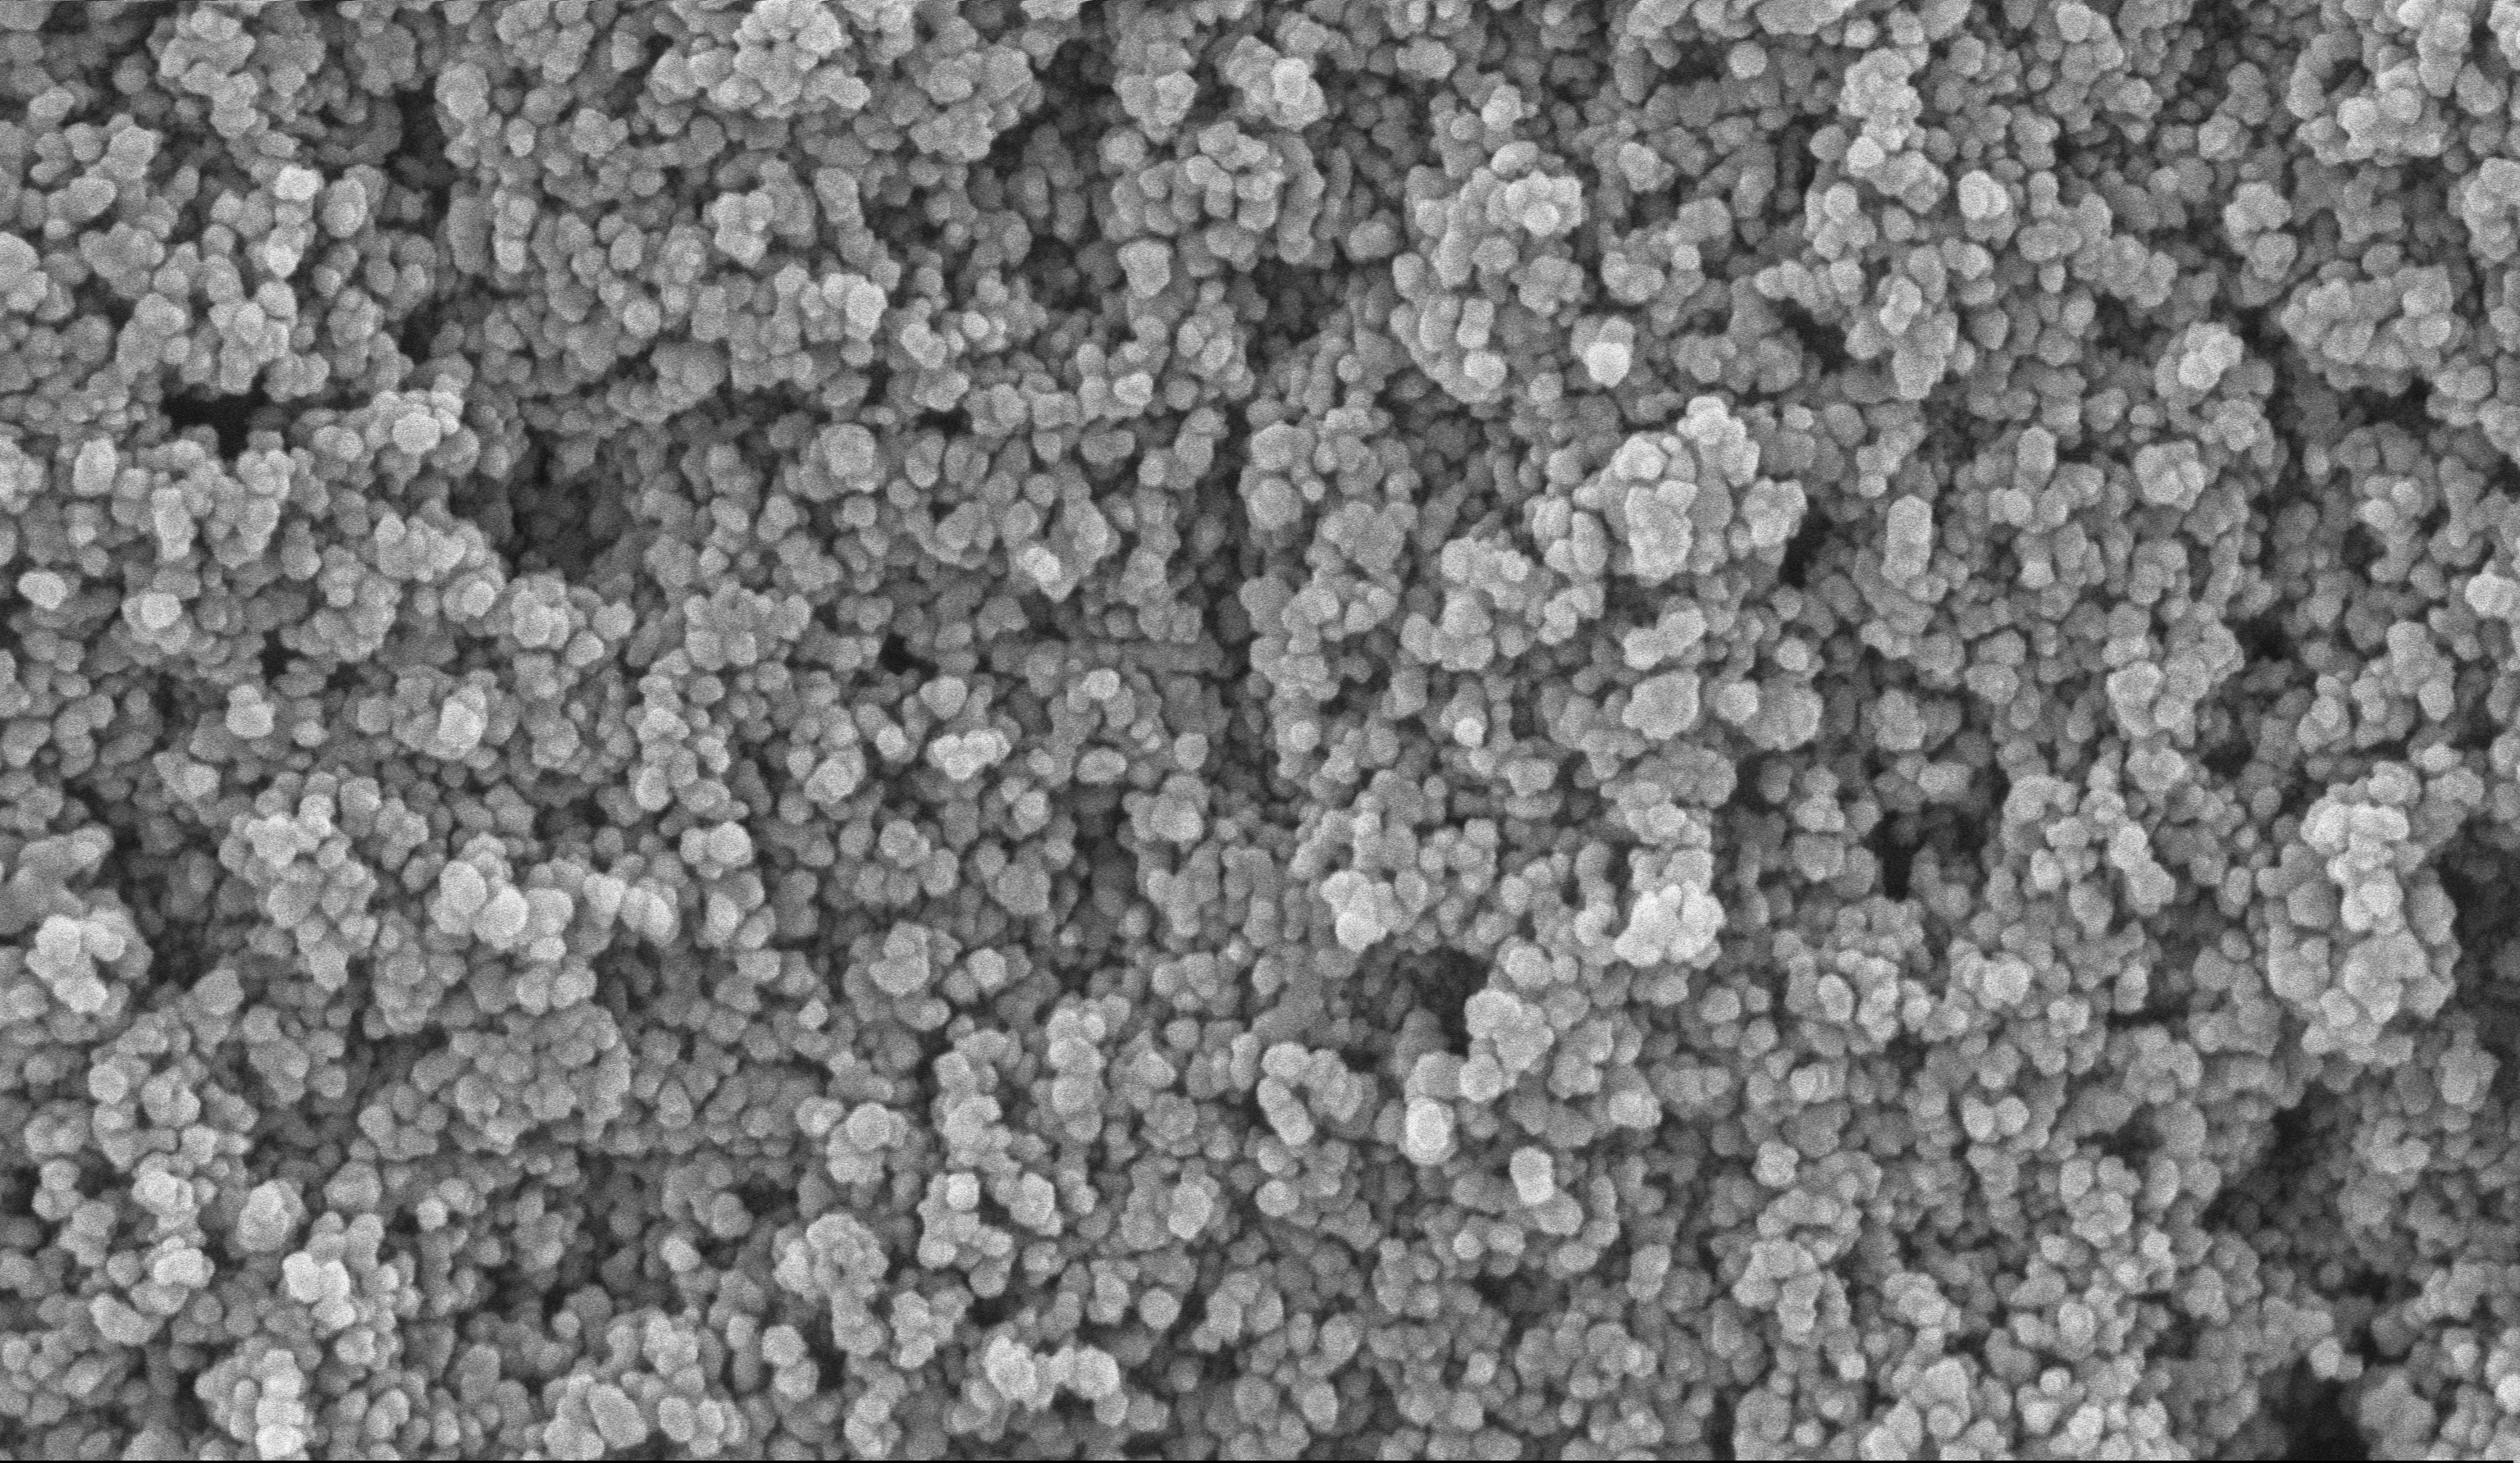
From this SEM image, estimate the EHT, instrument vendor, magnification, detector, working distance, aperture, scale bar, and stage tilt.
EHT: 10 kV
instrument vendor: Zeiss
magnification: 135 K X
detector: InLens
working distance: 5.1 mm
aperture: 30 µm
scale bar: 200 nm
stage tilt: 0°